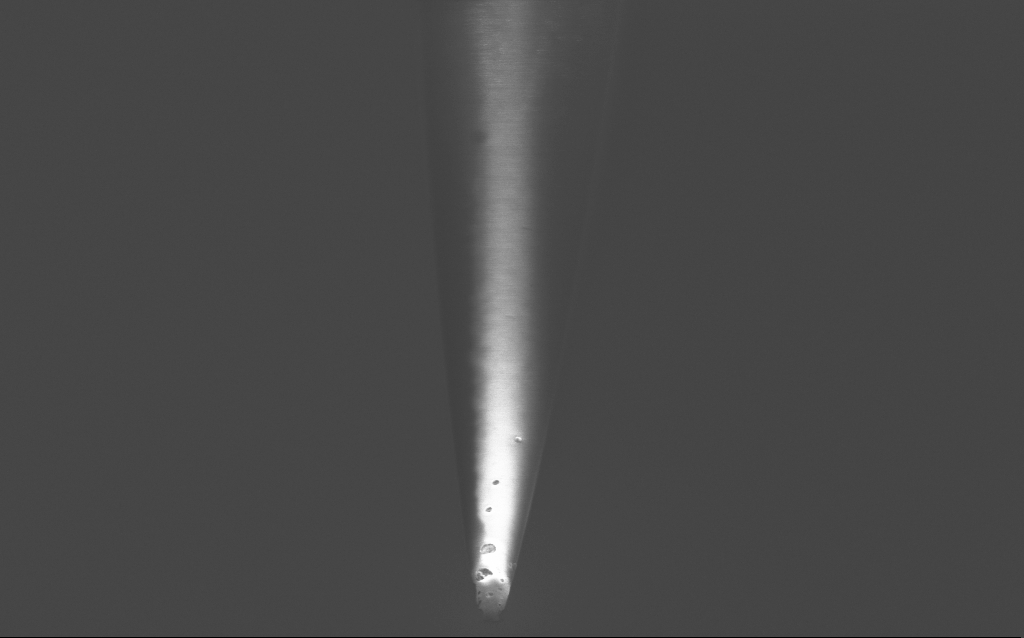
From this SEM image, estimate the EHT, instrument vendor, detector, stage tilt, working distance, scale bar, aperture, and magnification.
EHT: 2 kV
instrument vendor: Zeiss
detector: InLens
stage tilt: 45°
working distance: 6 mm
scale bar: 2000 nm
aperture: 30 µm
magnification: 28.28 K X